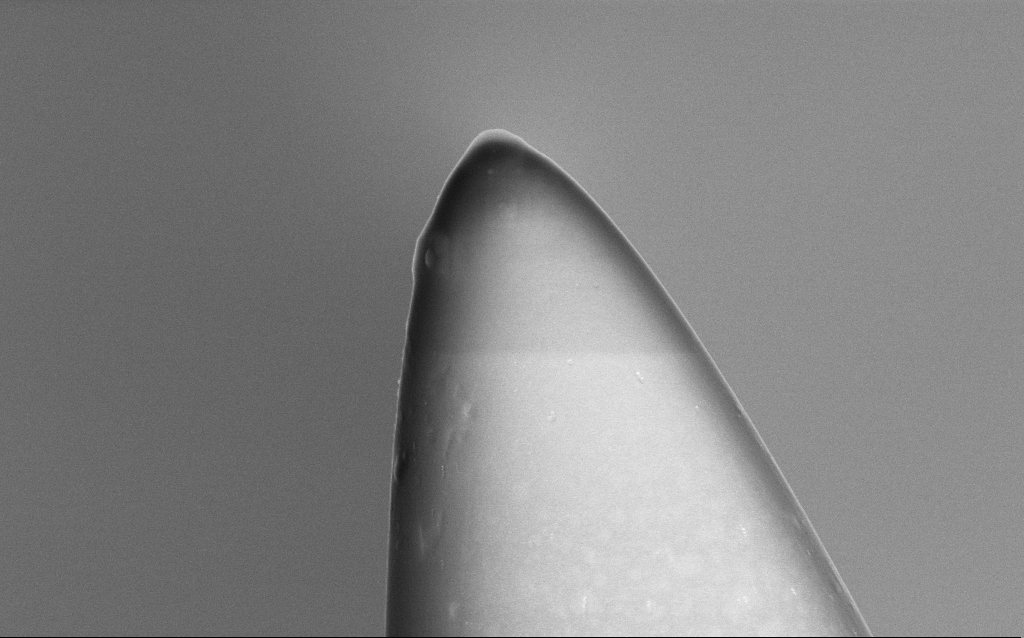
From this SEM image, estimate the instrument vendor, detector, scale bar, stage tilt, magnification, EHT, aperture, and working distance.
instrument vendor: Zeiss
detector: InLens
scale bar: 1000 nm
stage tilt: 0°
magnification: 54.92 K X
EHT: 5 kV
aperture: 30 µm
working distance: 3.3 mm